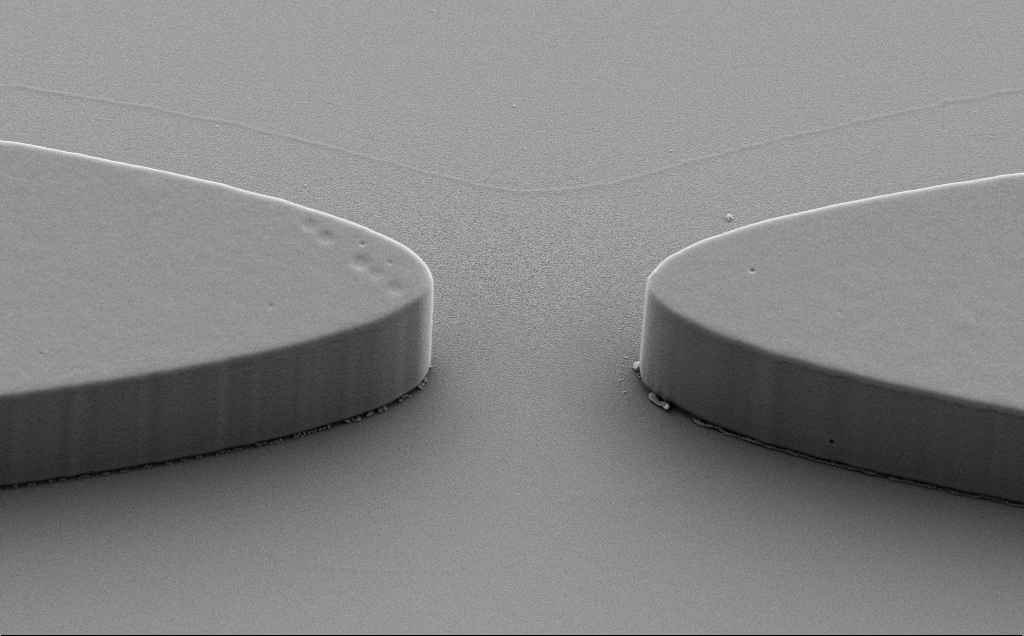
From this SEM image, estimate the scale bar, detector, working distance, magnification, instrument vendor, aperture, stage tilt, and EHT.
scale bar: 10000 nm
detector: SE2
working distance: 8 mm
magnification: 6.82 K X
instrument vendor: Zeiss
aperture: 30 µm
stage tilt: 40°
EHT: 5 kV